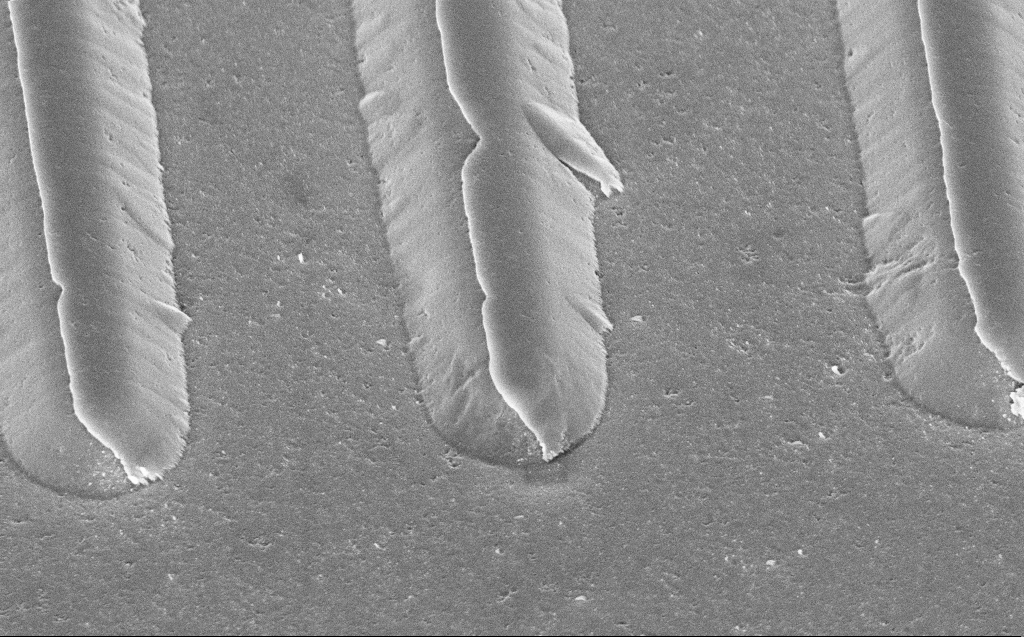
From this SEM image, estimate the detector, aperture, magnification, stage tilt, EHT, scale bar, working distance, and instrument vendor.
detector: InLens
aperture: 30 µm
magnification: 17.21 K X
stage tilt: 45°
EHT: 5 kV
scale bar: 2000 nm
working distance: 11 mm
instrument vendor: Zeiss